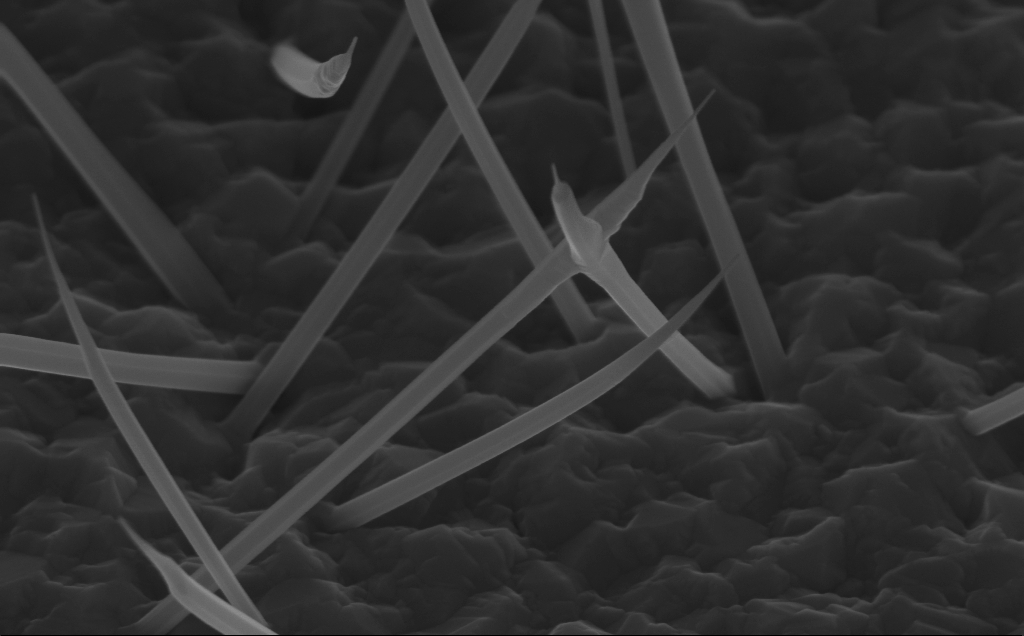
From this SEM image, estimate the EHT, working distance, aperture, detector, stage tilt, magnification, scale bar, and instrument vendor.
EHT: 10 kV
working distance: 5 mm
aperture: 30 µm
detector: InLens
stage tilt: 45°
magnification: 97.13 K X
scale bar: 200 nm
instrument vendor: Zeiss